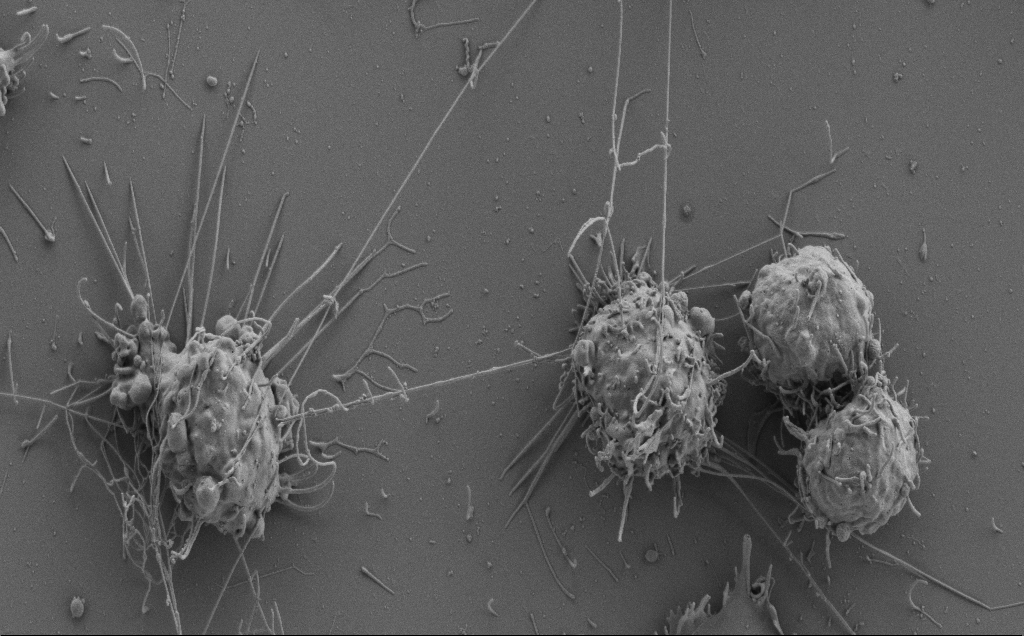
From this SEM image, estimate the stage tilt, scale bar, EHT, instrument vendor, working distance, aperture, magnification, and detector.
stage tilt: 0.8°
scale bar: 10000 nm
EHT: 3 kV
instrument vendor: Zeiss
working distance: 6 mm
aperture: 30 µm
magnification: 6.16 K X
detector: SE2